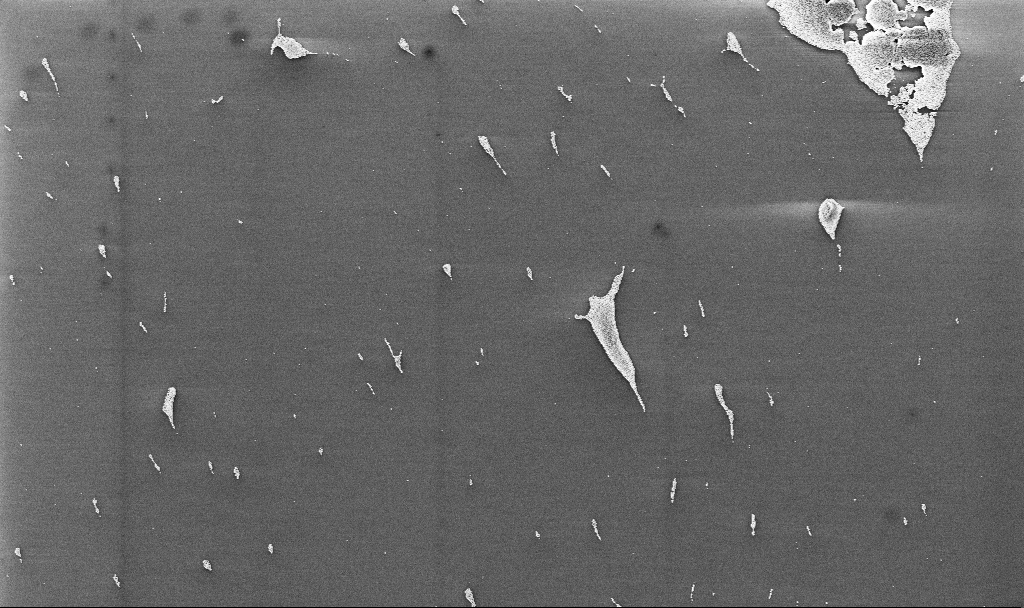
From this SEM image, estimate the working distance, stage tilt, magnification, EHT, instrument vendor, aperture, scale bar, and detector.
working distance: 3.1 mm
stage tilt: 0°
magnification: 12.71 K X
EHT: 4 kV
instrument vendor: Zeiss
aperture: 30 µm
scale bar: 1000 nm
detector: InLens